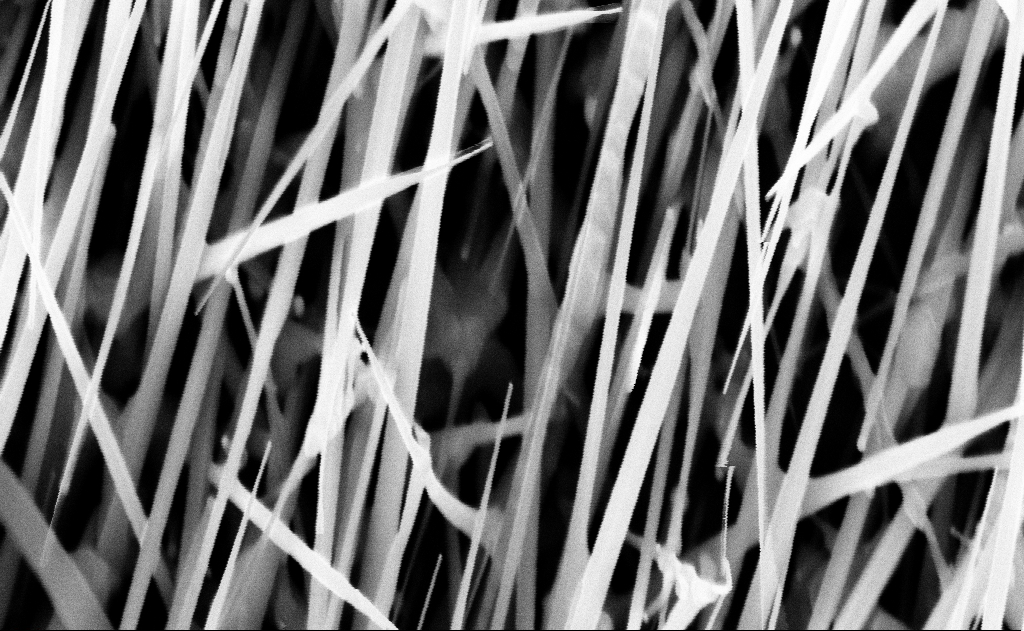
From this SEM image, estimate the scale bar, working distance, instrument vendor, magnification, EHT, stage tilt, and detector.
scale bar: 200 nm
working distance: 16 mm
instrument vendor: Zeiss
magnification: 80 K X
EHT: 10 kV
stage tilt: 0°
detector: InLens